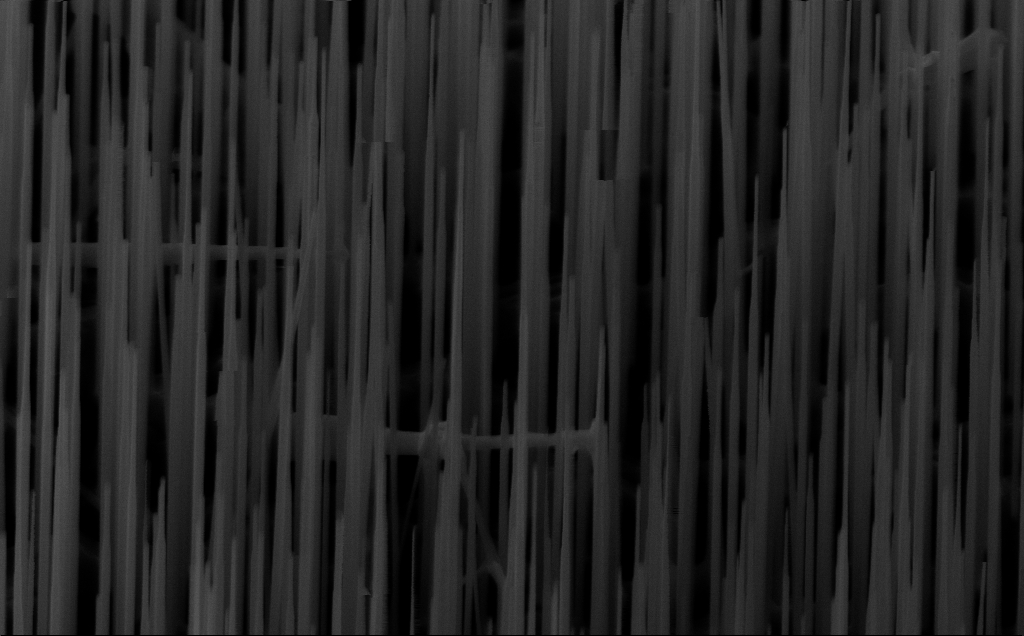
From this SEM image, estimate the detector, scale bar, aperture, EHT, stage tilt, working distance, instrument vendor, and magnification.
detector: InLens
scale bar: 1000 nm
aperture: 30 µm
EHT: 10 kV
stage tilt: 45°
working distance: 6 mm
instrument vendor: Zeiss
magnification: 40 K X